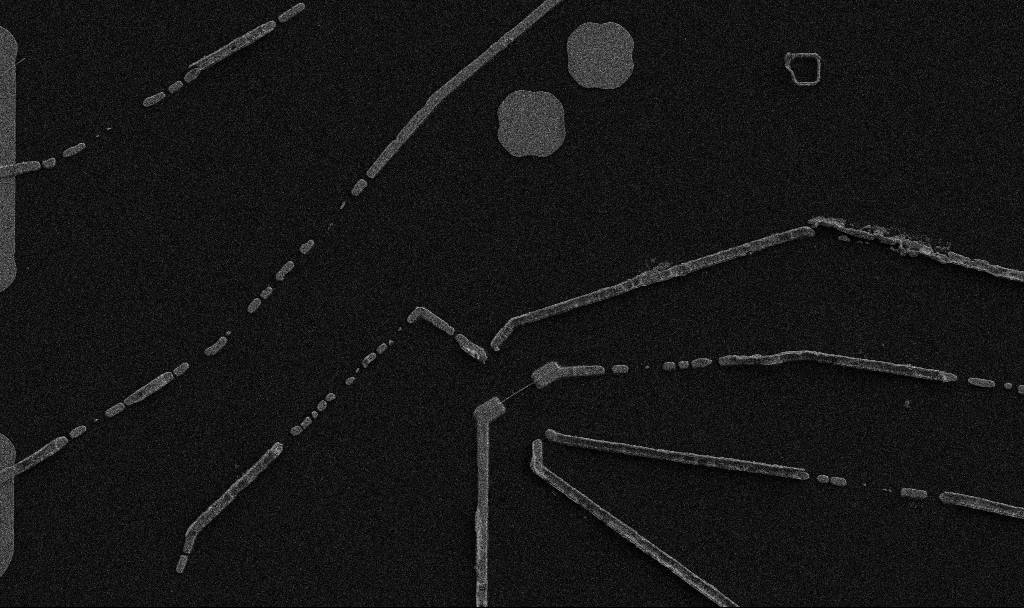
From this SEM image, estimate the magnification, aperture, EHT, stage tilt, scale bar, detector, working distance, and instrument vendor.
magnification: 5 K X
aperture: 30 µm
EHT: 5 kV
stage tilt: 0°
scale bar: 10000 nm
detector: SE2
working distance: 10.7 mm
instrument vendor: Zeiss